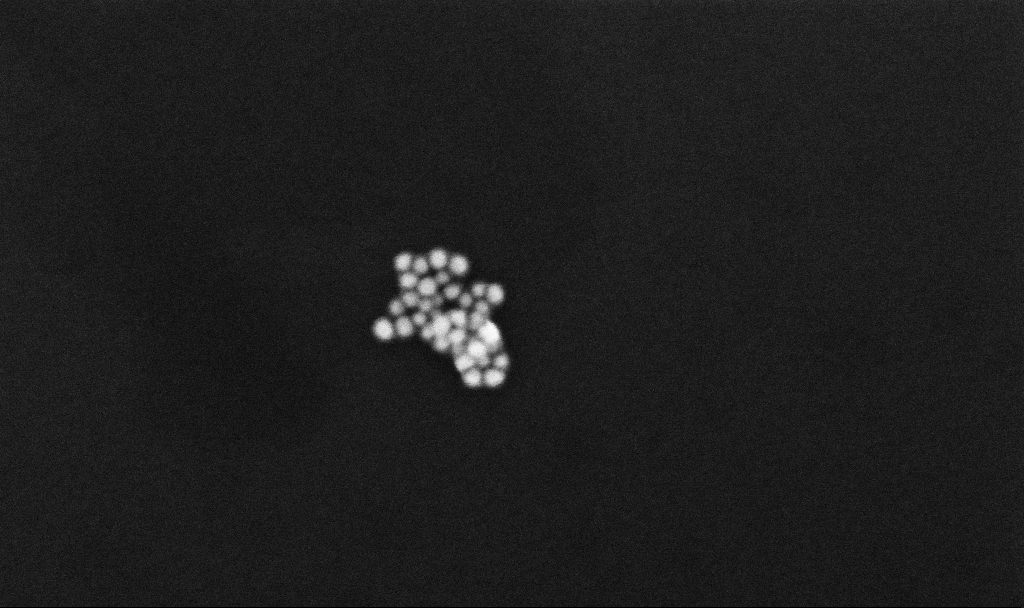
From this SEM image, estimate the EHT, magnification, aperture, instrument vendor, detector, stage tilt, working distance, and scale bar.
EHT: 10 kV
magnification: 290.79 K X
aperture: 30 µm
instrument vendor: Zeiss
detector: InLens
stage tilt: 0°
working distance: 3.3 mm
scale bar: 200 nm